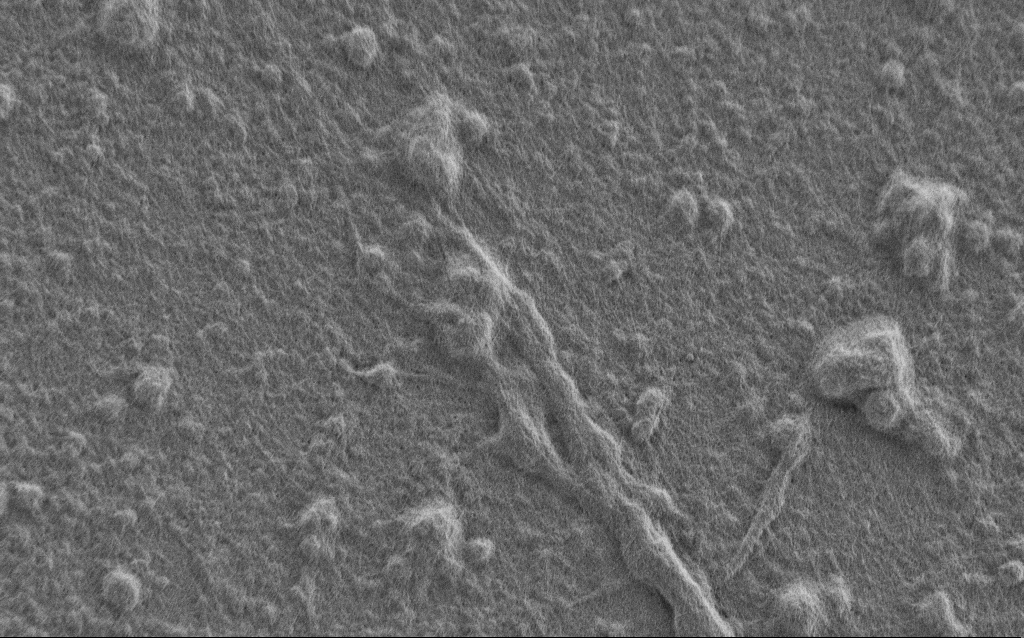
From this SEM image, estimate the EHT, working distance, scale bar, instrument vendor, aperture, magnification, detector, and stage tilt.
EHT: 0.9 kV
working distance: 7 mm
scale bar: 2000 nm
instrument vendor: Zeiss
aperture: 30 µm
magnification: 7.5 K X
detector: SE2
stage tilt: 0°